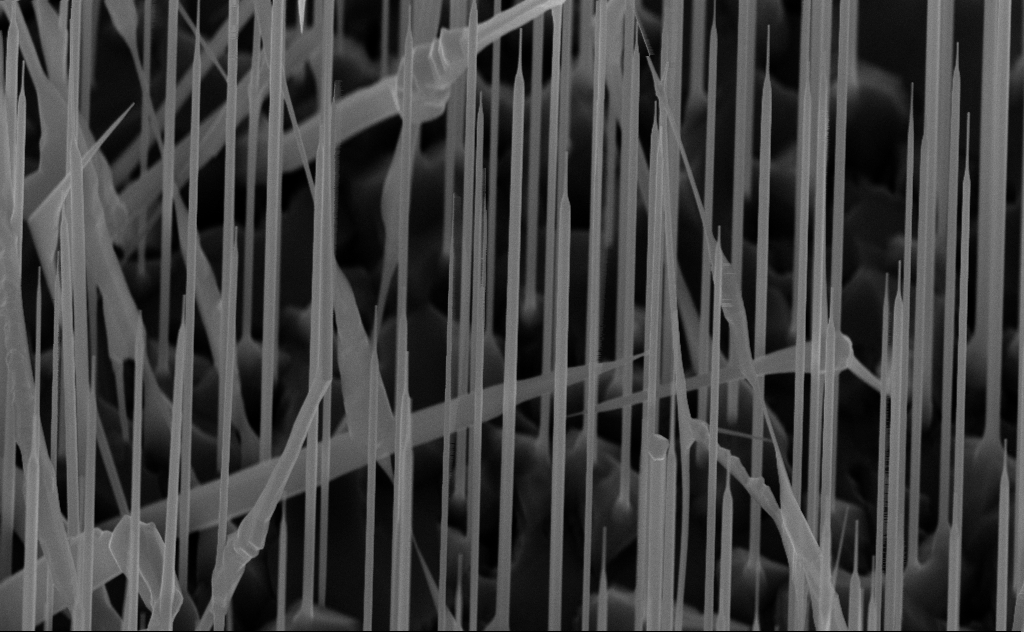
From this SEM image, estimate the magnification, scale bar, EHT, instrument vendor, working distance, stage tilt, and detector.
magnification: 40 K X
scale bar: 1000 nm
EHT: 10 kV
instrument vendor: Zeiss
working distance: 7 mm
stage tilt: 44.9°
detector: InLens